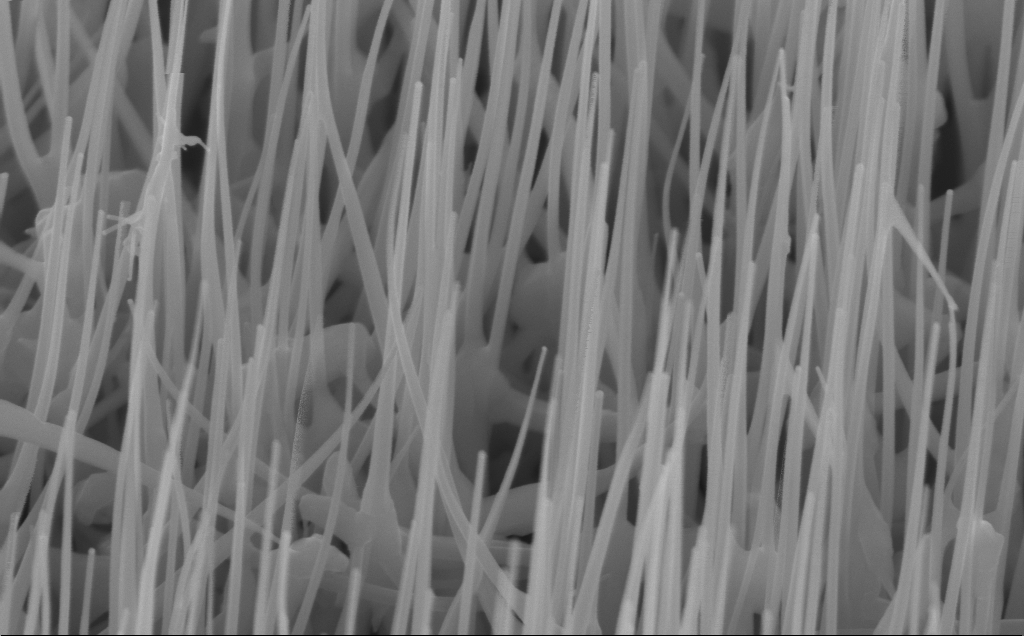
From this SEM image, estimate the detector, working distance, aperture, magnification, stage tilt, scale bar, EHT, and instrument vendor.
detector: InLens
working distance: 6 mm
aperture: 30 µm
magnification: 100 K X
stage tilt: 45°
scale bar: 200 nm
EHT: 10 kV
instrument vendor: Zeiss